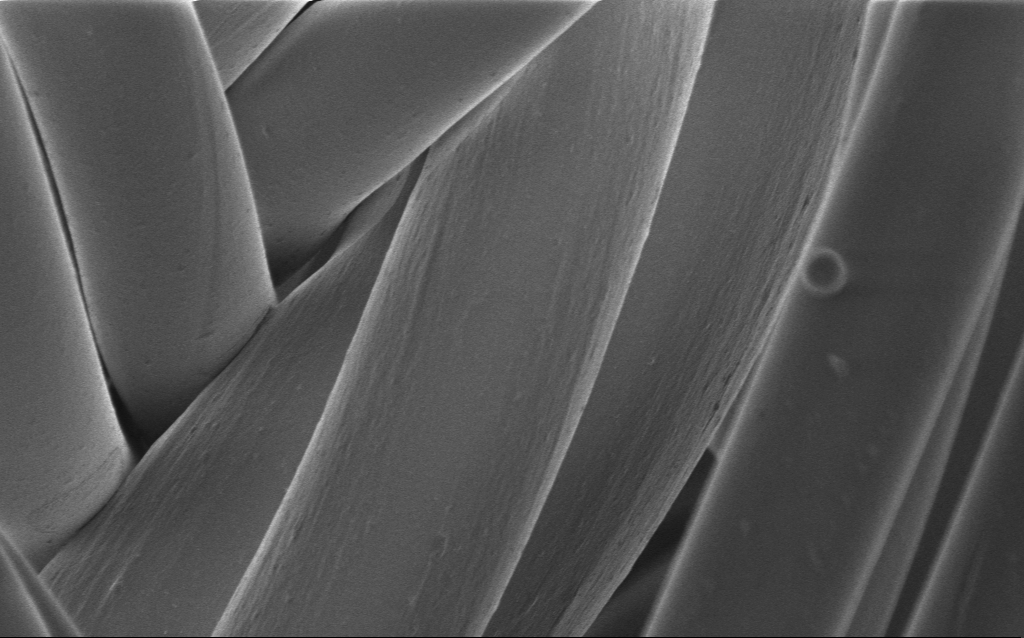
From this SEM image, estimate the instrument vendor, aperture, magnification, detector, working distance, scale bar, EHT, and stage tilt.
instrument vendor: Zeiss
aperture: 30 µm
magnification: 2.9 K X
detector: InLens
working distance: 4 mm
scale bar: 10000 nm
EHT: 1 kV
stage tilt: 0°